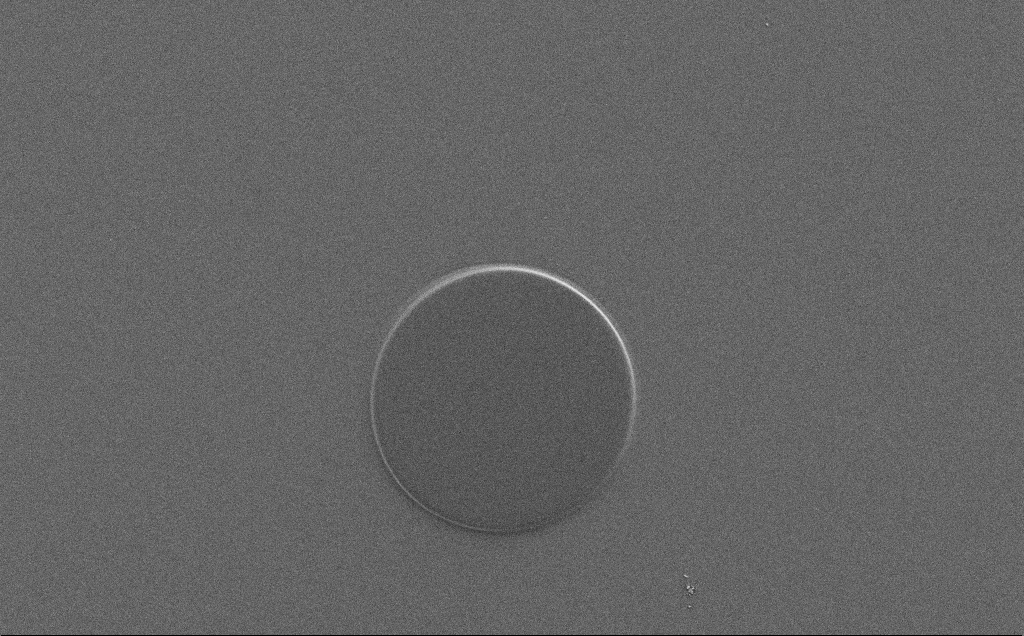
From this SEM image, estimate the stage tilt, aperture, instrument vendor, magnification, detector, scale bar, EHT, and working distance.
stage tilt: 0°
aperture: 30 µm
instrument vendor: Zeiss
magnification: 1.63 K X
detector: SE2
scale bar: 20000 nm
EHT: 1.5 kV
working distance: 4 mm